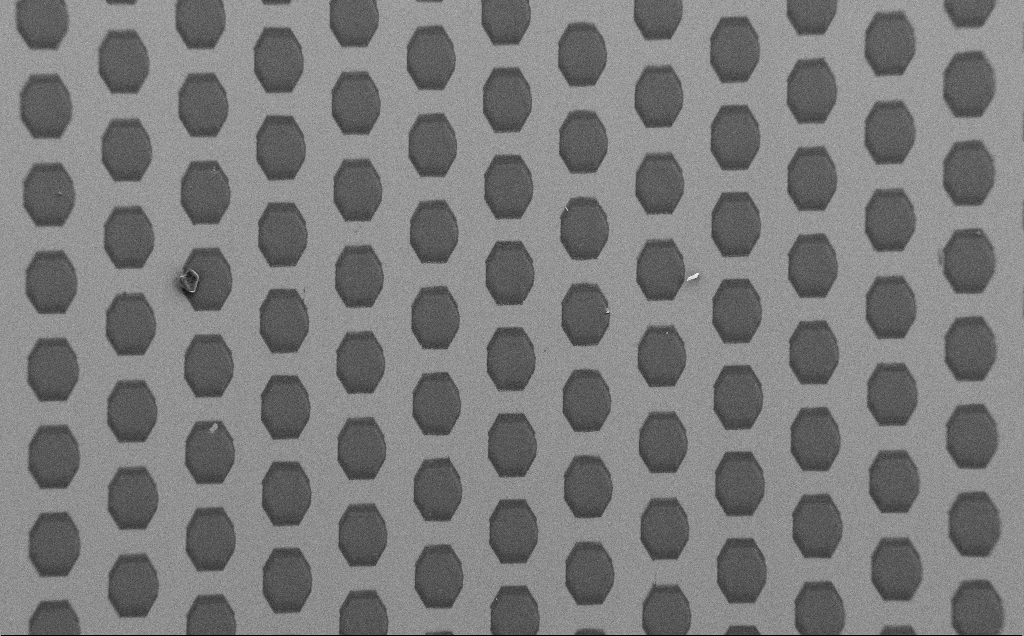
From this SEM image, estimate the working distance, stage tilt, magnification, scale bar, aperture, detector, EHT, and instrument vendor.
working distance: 6 mm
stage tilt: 0°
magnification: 0.318 K X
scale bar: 100000 nm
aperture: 30 µm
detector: SE2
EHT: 1.5 kV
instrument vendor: Zeiss